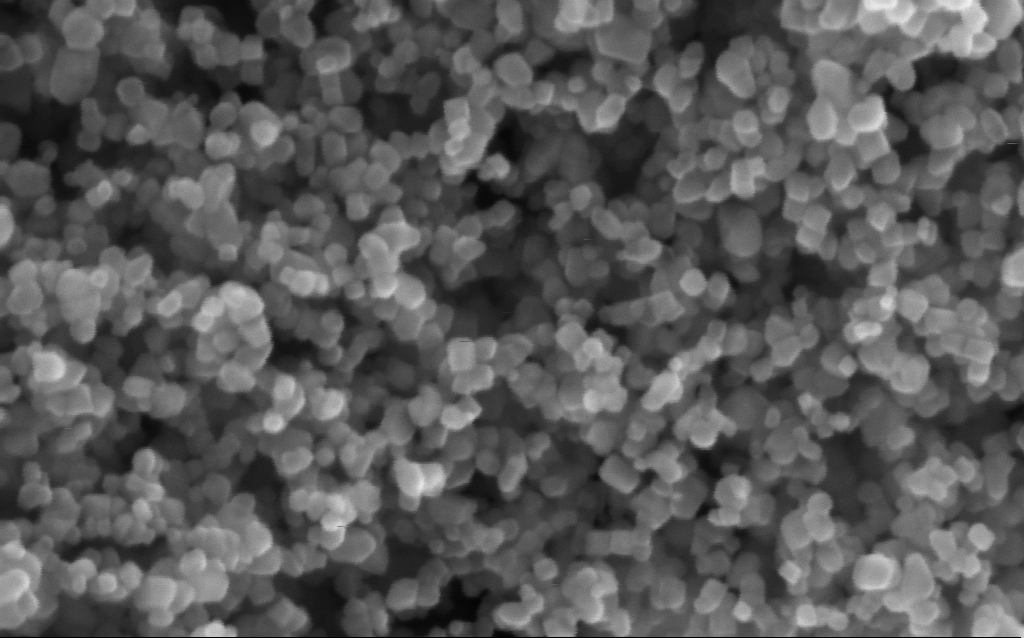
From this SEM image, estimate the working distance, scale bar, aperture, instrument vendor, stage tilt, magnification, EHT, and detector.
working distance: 3 mm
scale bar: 100 nm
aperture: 30 µm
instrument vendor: Zeiss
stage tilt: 0°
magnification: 416 K X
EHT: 5 kV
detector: InLens